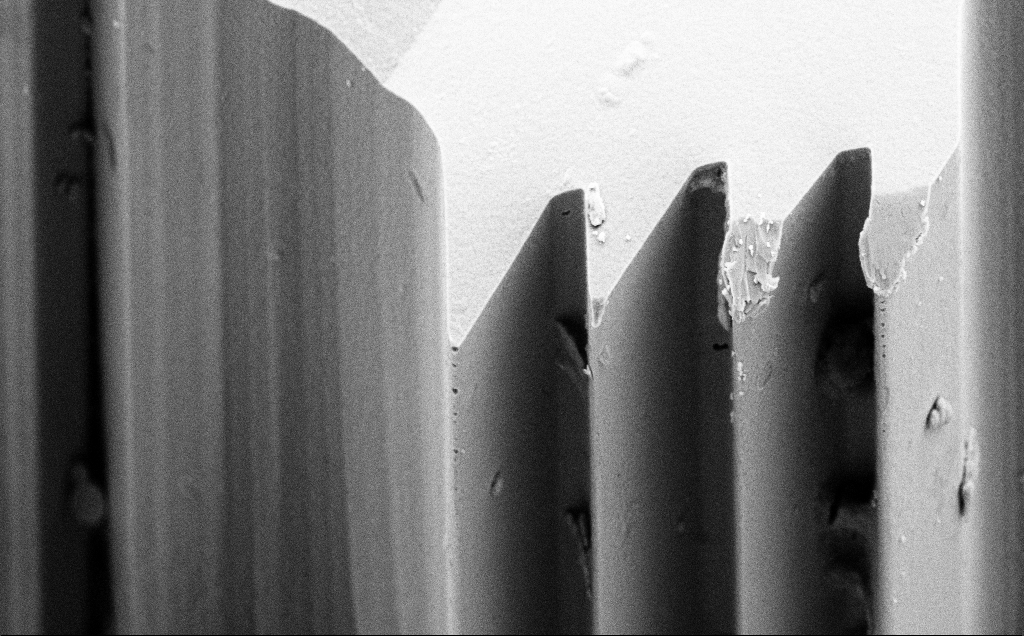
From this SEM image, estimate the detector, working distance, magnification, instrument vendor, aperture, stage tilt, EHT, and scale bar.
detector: SE2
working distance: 8 mm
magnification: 4.6 K X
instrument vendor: Zeiss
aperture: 30 µm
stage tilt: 45°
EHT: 10 kV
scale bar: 10000 nm